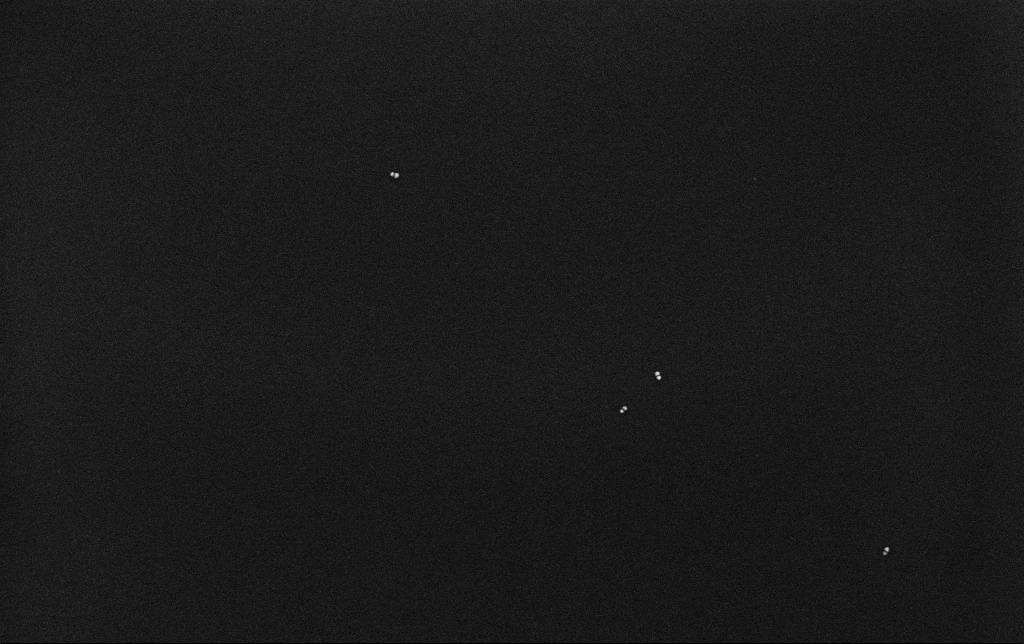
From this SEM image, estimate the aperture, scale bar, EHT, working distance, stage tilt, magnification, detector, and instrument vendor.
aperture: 30 µm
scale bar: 200 nm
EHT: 10 kV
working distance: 3.2 mm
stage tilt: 0°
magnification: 100 K X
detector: InLens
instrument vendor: Zeiss